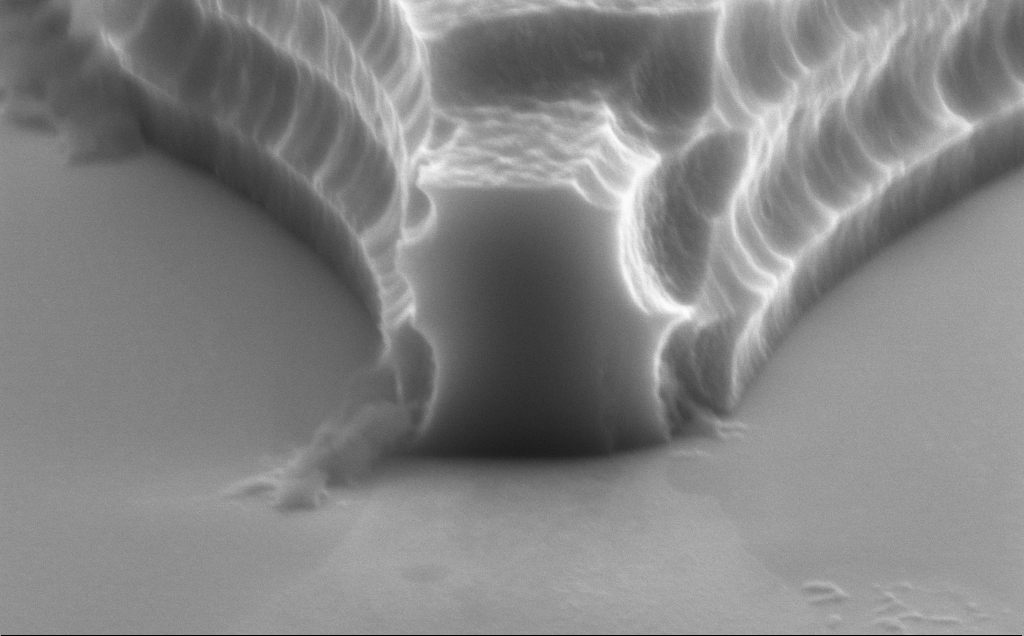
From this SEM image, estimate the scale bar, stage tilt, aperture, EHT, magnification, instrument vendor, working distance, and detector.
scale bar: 1000 nm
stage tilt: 70°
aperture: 30 µm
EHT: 8 kV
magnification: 62.61 K X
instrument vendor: Zeiss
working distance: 12 mm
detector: SE2